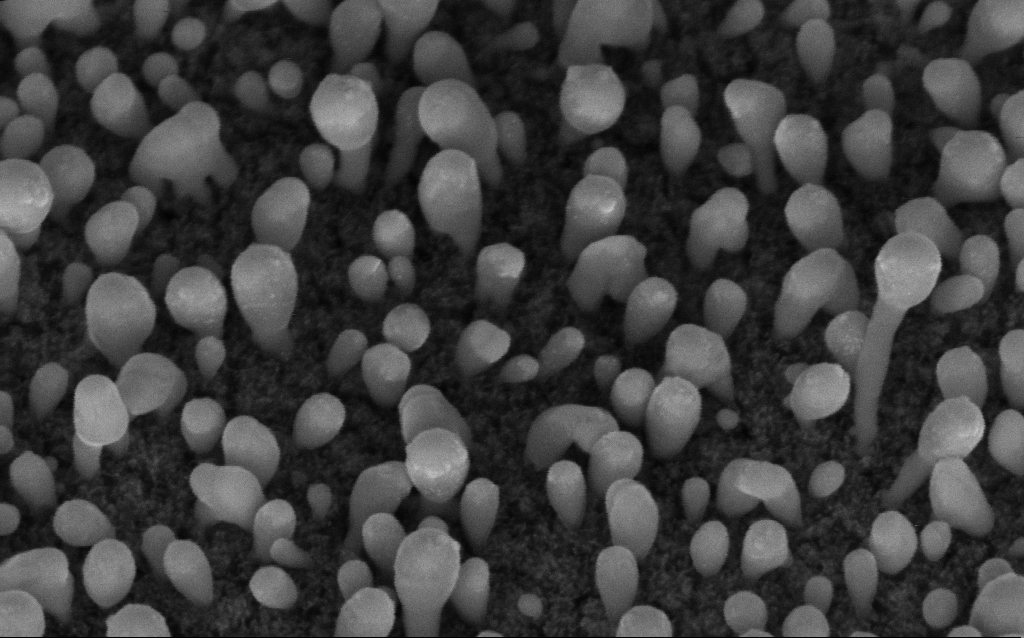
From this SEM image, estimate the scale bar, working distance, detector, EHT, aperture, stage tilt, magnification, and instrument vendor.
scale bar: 100 nm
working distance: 6.2 mm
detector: InLens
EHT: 5 kV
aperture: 30 µm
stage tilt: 45°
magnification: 200 K X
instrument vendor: Zeiss